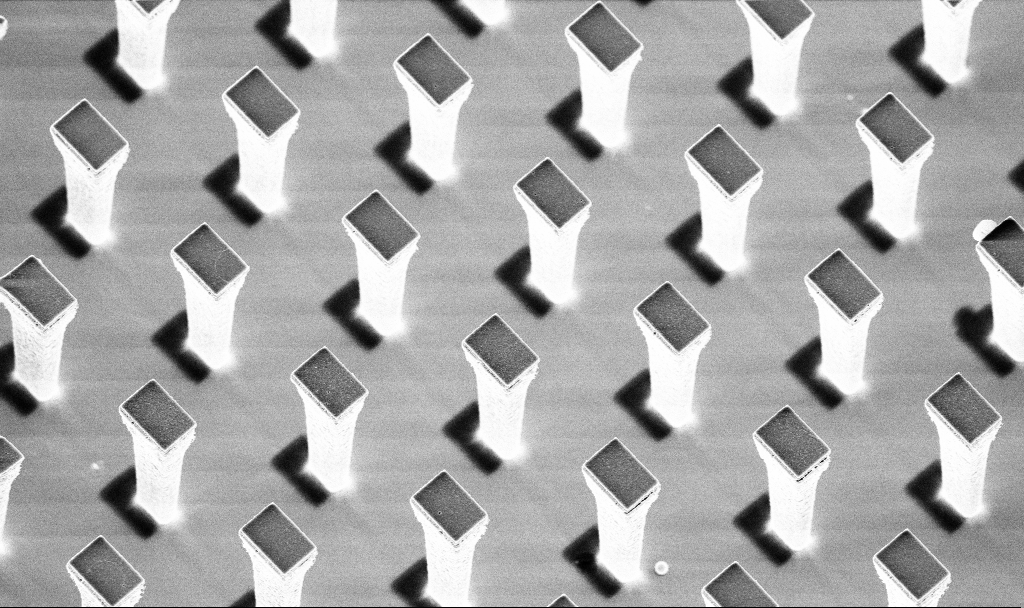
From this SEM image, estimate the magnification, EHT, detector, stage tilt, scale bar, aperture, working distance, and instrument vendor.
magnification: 5.5 K X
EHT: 5 kV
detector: InLens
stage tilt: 20°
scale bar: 10000 nm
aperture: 30 µm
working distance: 3.3 mm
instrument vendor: Zeiss